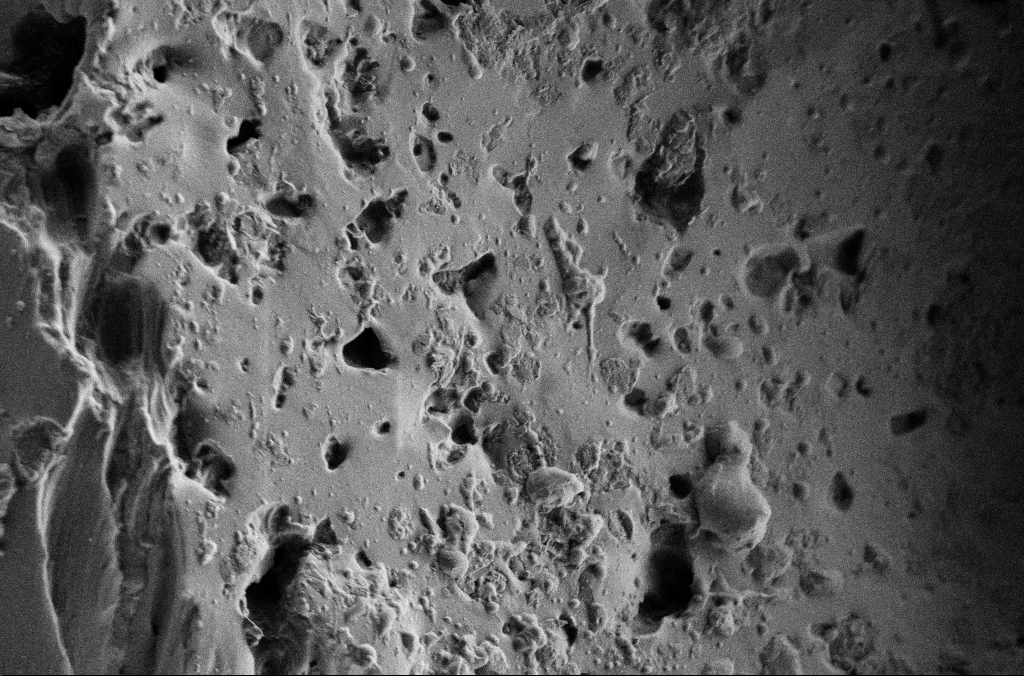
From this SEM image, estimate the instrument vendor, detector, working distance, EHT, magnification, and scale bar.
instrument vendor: Zeiss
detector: SE2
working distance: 3 mm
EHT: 2 kV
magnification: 5 K X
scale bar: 10000 nm